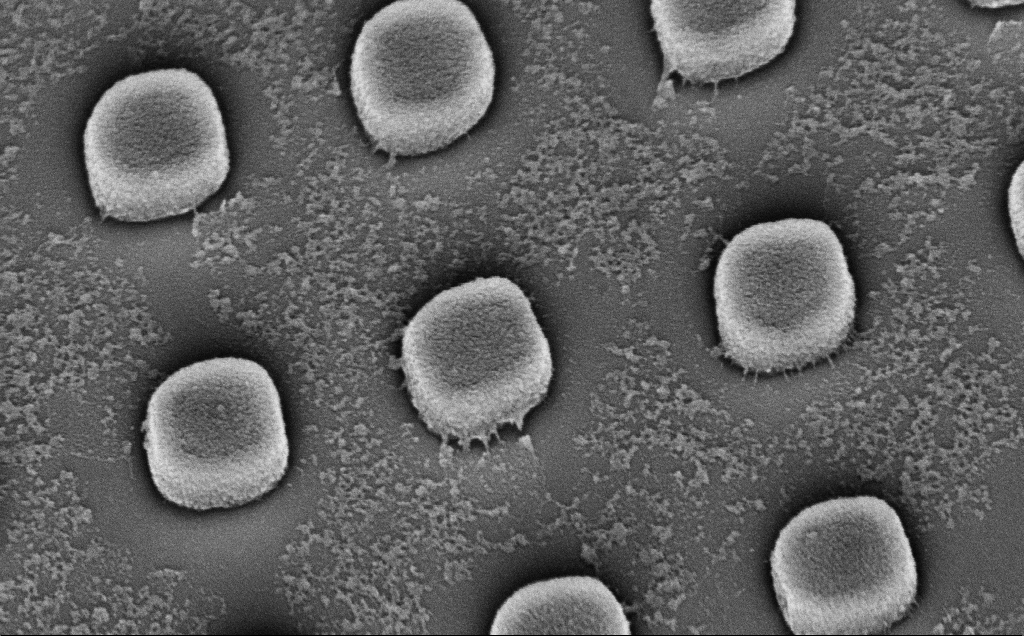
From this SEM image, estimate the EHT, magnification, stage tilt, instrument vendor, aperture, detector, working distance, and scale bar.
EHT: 5 kV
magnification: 72.75 K X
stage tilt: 45°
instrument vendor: Zeiss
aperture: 30 µm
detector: InLens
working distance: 7 mm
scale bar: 200 nm